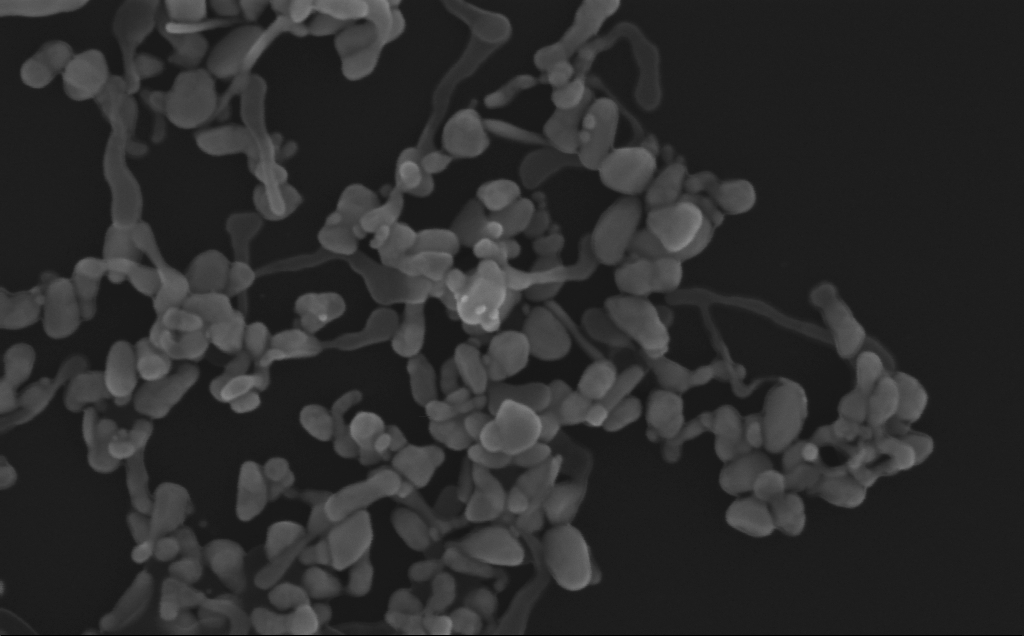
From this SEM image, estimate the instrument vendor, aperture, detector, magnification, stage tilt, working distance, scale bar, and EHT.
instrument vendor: Zeiss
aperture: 30 µm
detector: InLens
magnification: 226.71 K X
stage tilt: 0°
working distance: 3 mm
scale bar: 200 nm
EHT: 10 kV